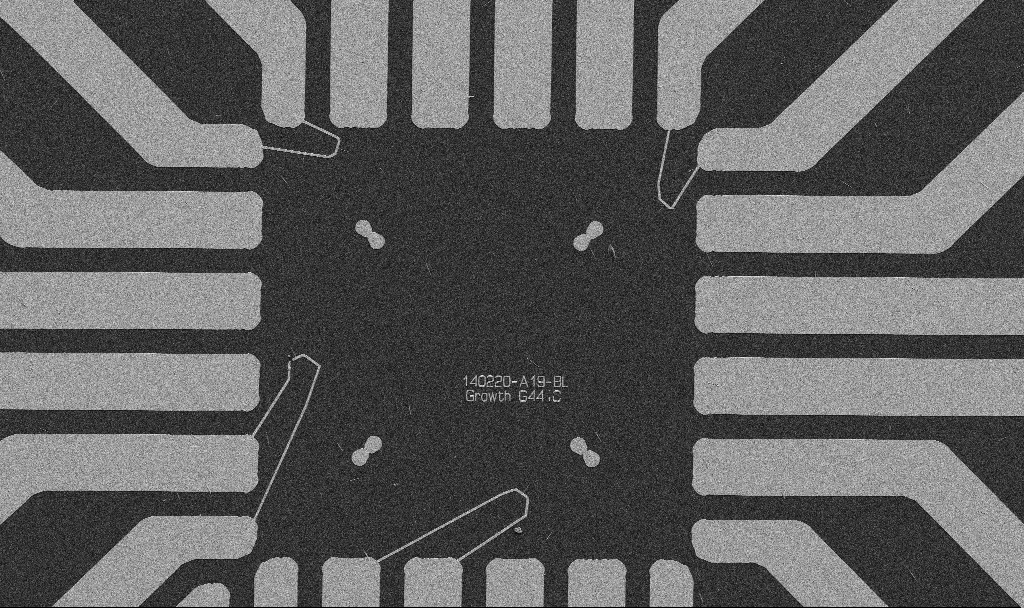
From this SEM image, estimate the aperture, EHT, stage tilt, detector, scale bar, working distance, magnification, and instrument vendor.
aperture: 30 µm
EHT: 5 kV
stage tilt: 0°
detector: SE2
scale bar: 20000 nm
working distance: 10.7 mm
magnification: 1 K X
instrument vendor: Zeiss